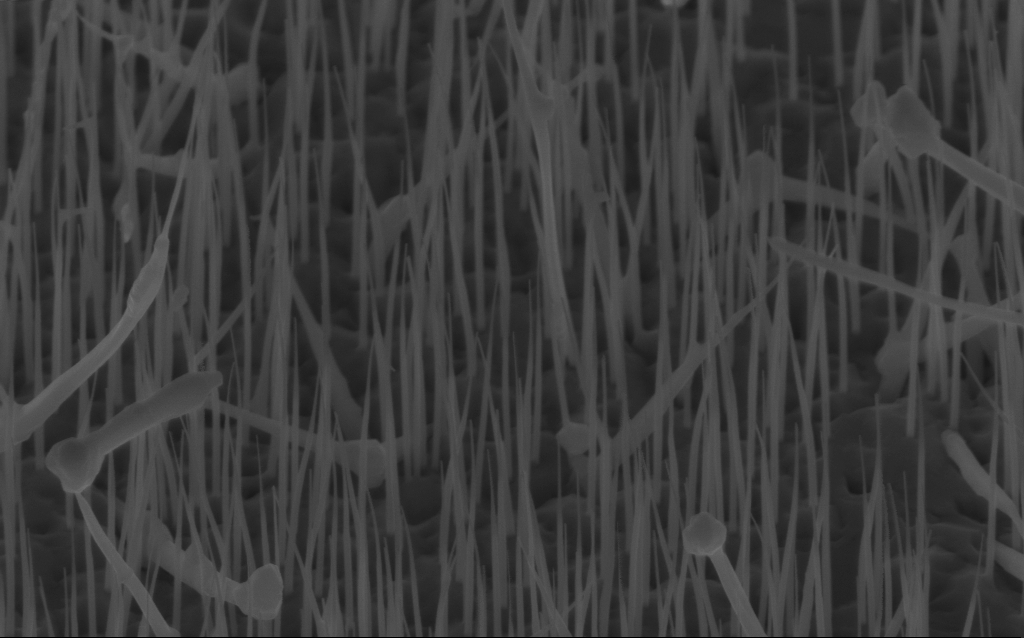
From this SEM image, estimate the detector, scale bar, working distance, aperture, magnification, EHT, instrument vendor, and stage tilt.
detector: InLens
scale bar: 1000 nm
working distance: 6 mm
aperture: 30 µm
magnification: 40 K X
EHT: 10 kV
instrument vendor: Zeiss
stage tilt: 45°